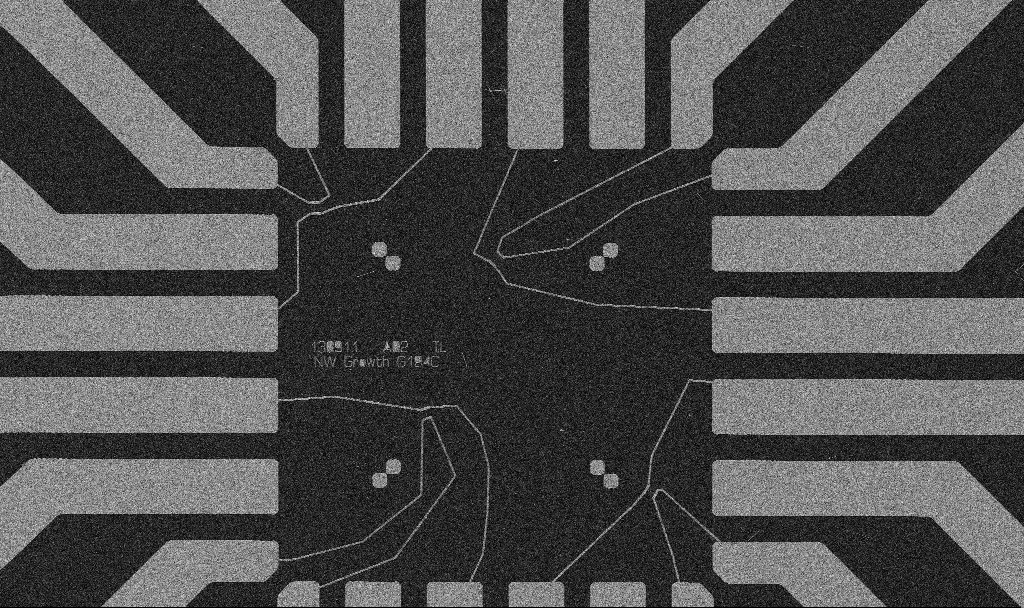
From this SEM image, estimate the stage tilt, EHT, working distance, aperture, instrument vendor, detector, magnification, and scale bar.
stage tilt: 0°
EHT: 5 kV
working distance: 10.7 mm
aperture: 30 µm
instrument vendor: Zeiss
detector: SE2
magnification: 1 K X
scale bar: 20000 nm